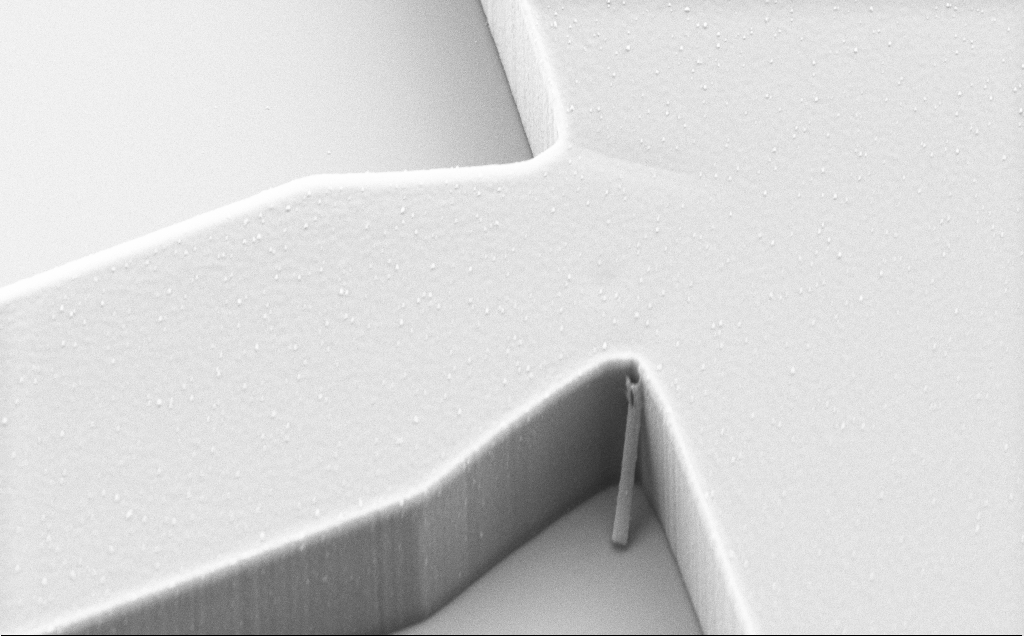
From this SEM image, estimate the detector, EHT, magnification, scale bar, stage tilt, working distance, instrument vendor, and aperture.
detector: SE2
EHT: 5 kV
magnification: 12.33 K X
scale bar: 2000 nm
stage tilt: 45°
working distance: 9 mm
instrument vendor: Zeiss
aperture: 30 µm